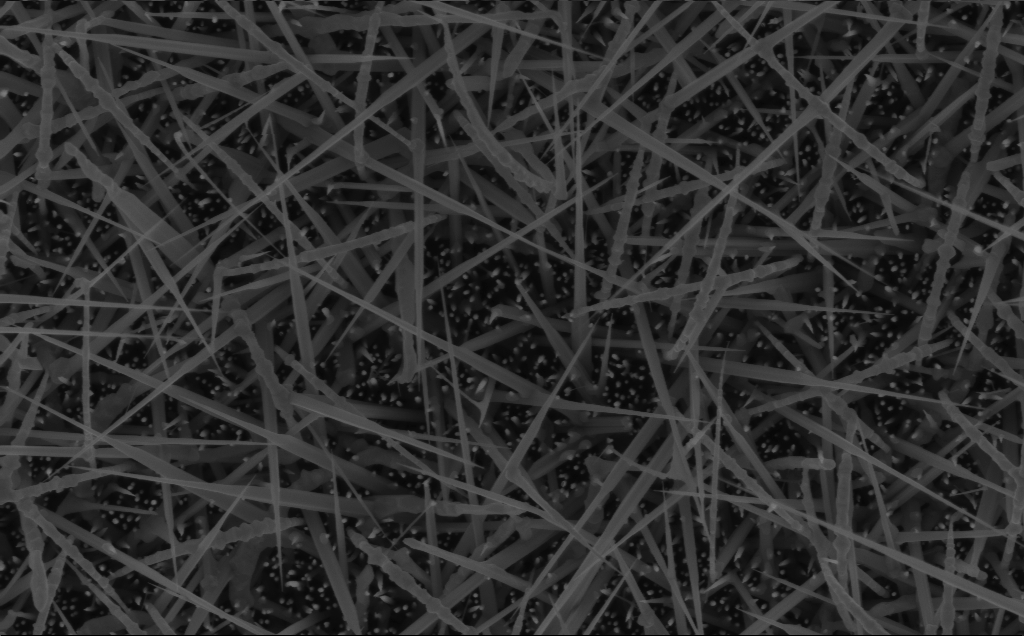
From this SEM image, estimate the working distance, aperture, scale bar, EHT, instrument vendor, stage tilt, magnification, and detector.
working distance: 6 mm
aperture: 30 µm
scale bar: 1000 nm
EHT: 5 kV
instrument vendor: Zeiss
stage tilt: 0°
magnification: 40 K X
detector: InLens